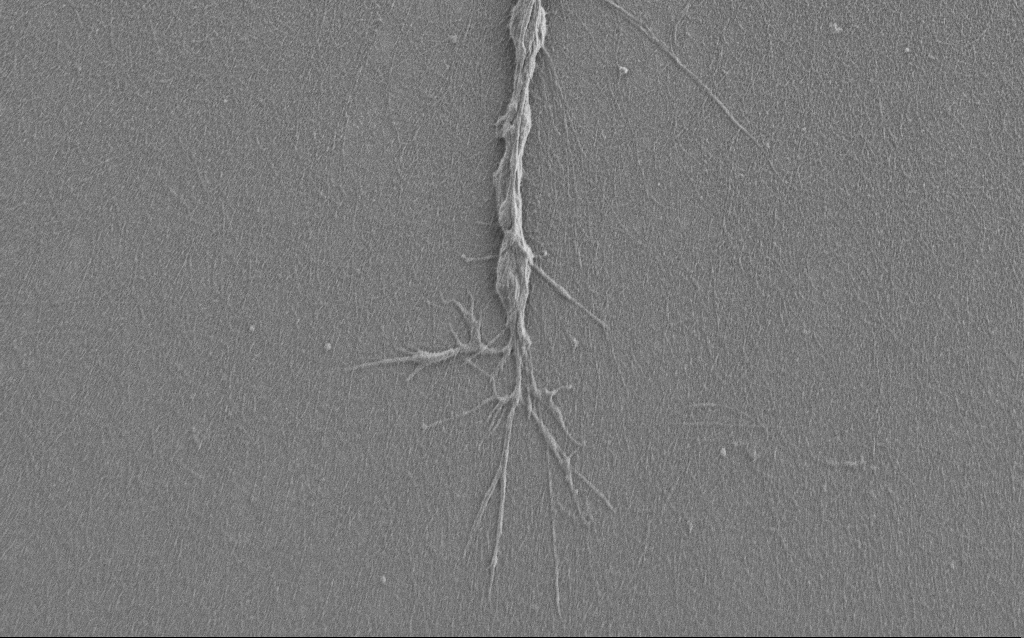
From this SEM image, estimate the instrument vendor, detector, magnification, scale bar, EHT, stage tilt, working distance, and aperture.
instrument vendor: Zeiss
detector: SE2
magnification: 7.5 K X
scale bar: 2000 nm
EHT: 1 kV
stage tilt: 0°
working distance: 6 mm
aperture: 30 µm